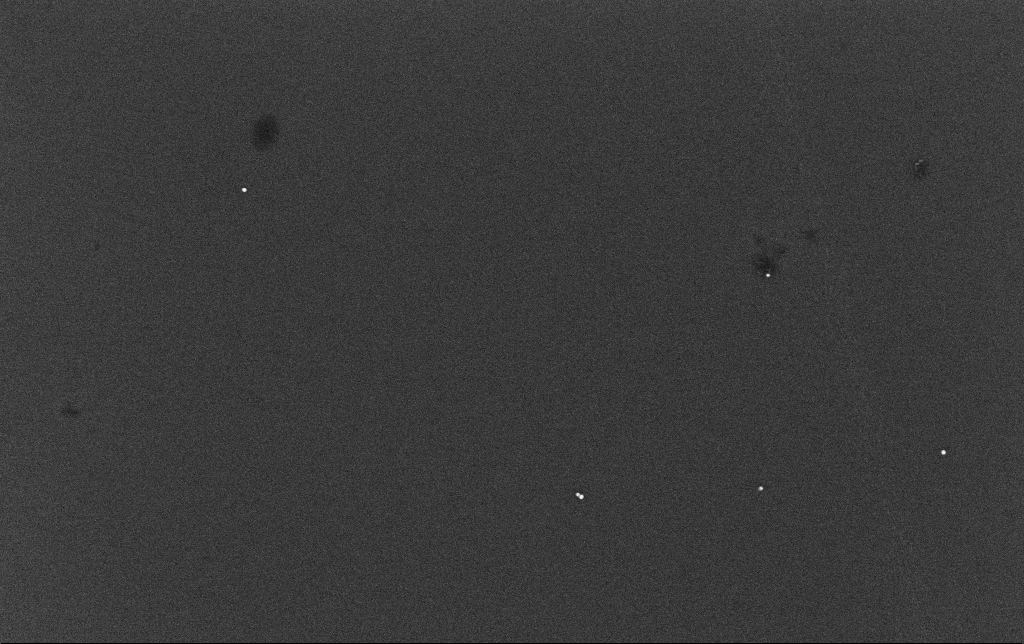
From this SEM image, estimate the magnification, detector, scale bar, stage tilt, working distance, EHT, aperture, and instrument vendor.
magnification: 100 K X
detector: InLens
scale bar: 200 nm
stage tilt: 0°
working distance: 3.4 mm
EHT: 10 kV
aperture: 30 µm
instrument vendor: Zeiss